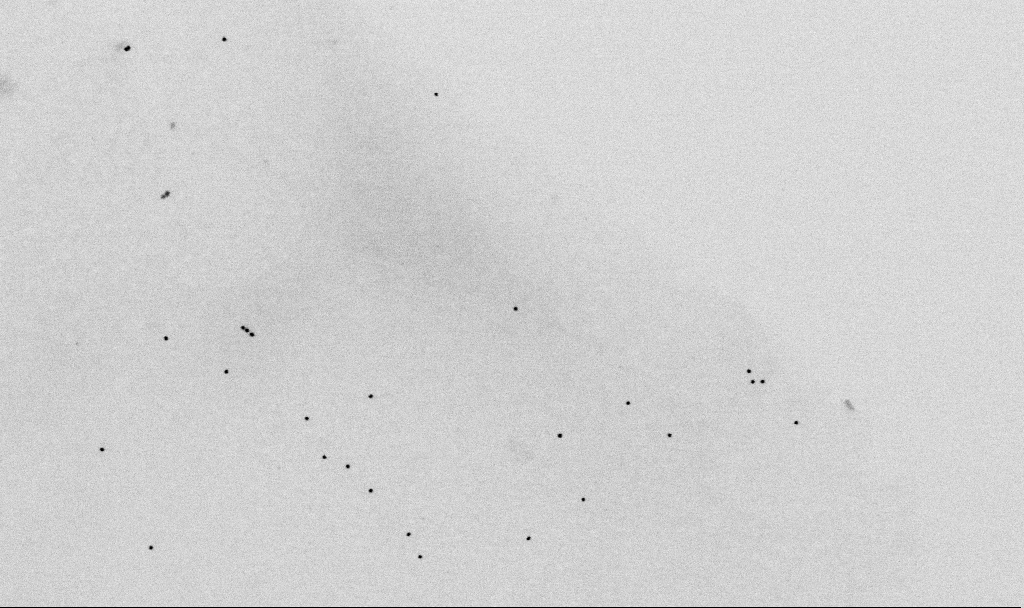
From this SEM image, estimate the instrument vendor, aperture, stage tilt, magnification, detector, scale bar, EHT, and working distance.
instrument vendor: Zeiss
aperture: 30 µm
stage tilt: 0°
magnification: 60 K X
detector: SE2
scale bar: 1000 nm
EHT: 2 kV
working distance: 5.5 mm